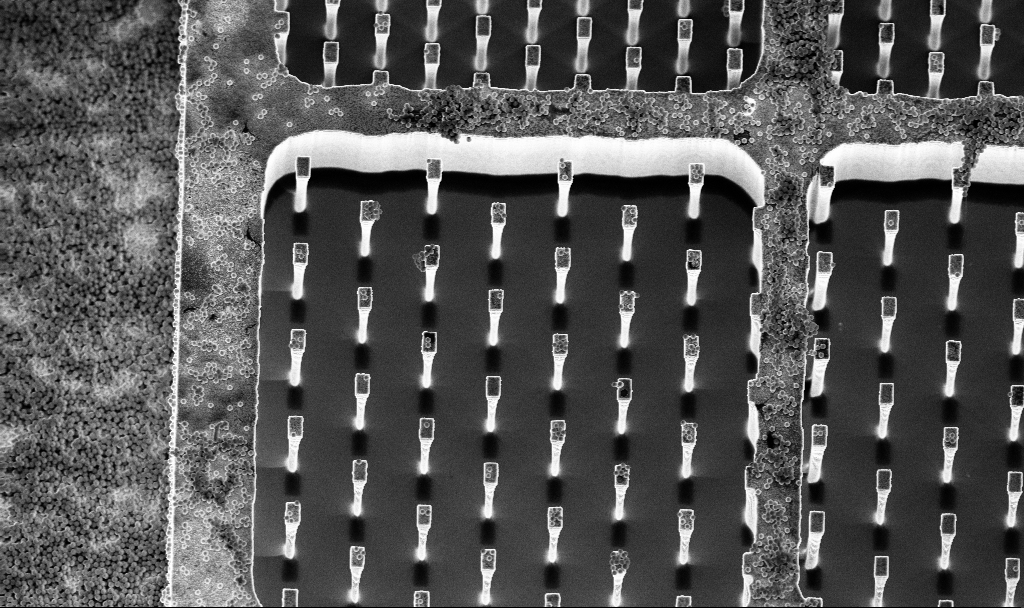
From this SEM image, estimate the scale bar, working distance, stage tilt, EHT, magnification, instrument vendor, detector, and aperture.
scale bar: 10000 nm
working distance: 3.2 mm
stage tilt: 15°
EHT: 5 kV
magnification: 3.39 K X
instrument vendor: Zeiss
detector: InLens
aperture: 30 µm